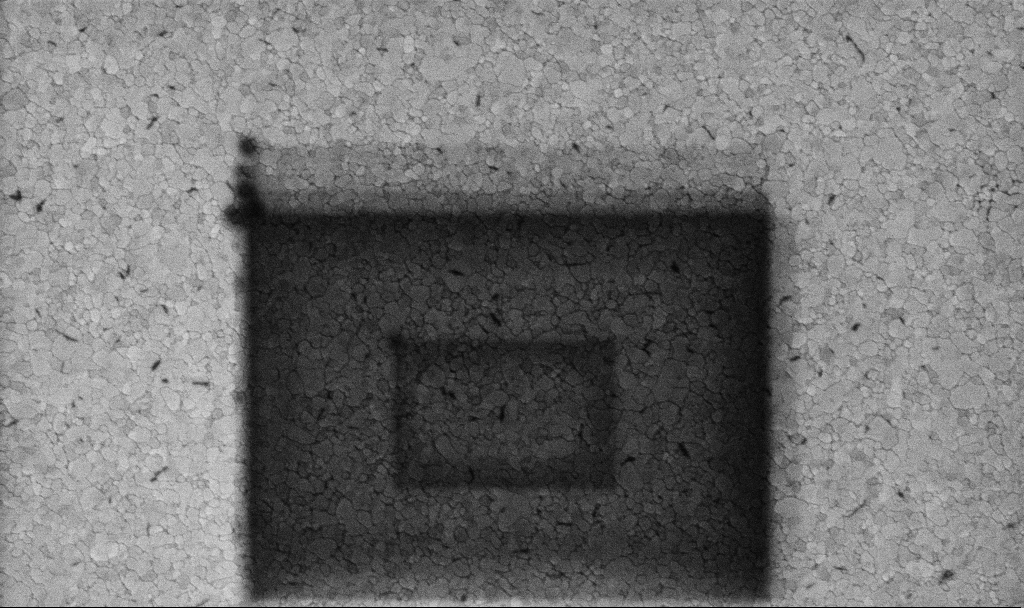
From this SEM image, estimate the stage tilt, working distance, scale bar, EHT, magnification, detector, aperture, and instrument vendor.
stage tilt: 0°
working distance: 3.1 mm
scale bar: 1000 nm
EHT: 5 kV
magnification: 50.15 K X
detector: InLens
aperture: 30 µm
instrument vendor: Zeiss